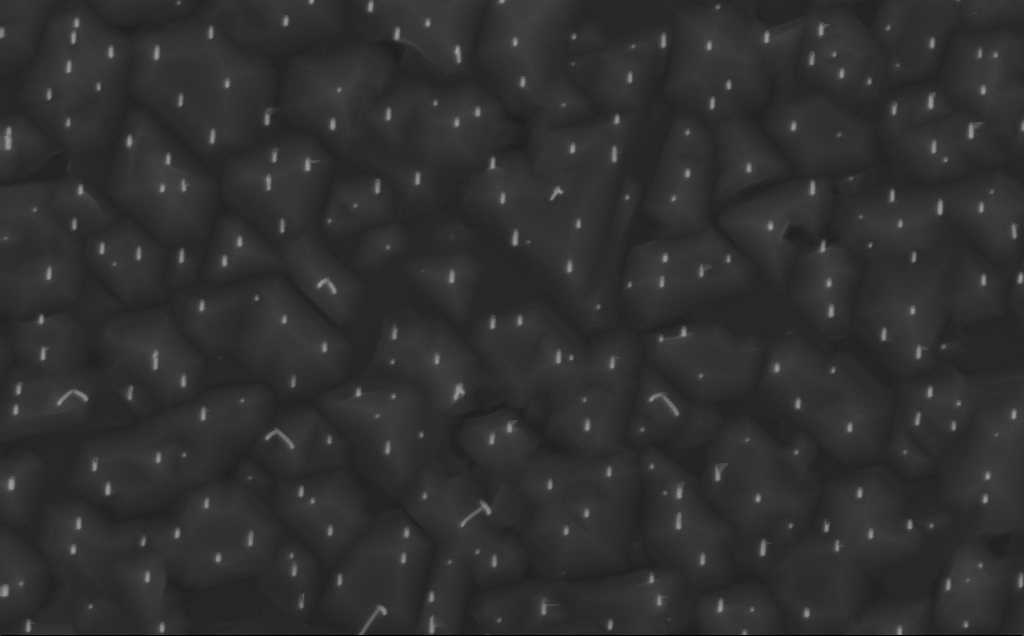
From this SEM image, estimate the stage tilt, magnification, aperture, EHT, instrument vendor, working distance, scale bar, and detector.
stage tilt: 0°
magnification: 40 K X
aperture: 30 µm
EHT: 10 kV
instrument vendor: Zeiss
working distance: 4 mm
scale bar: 1000 nm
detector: InLens